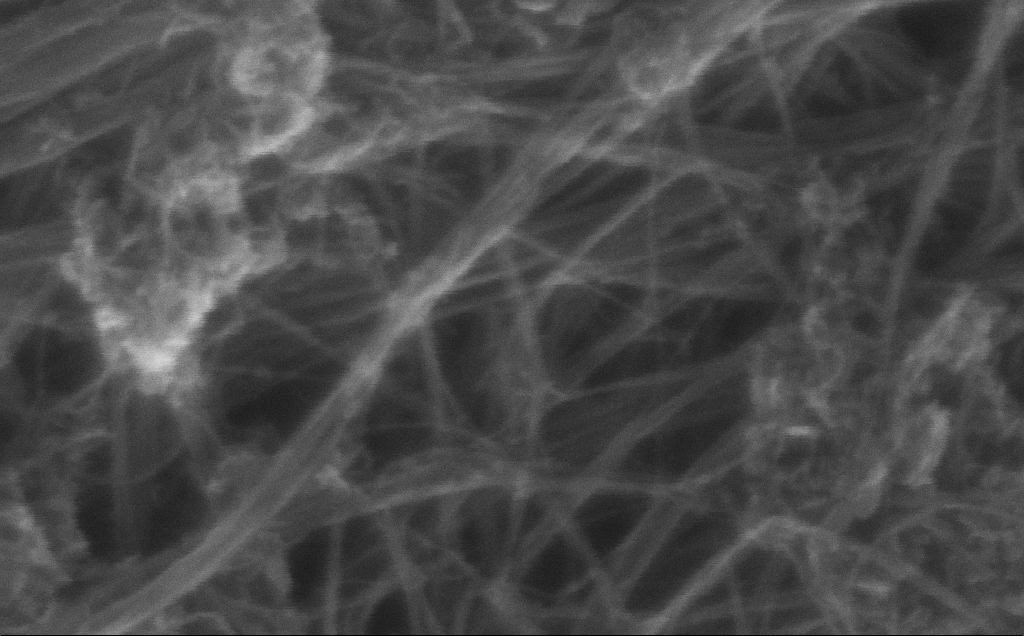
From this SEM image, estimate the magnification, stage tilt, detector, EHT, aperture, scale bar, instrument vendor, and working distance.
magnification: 539.31 K X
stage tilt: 0°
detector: InLens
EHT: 10 kV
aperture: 30 µm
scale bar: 100 nm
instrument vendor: Zeiss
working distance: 3 mm